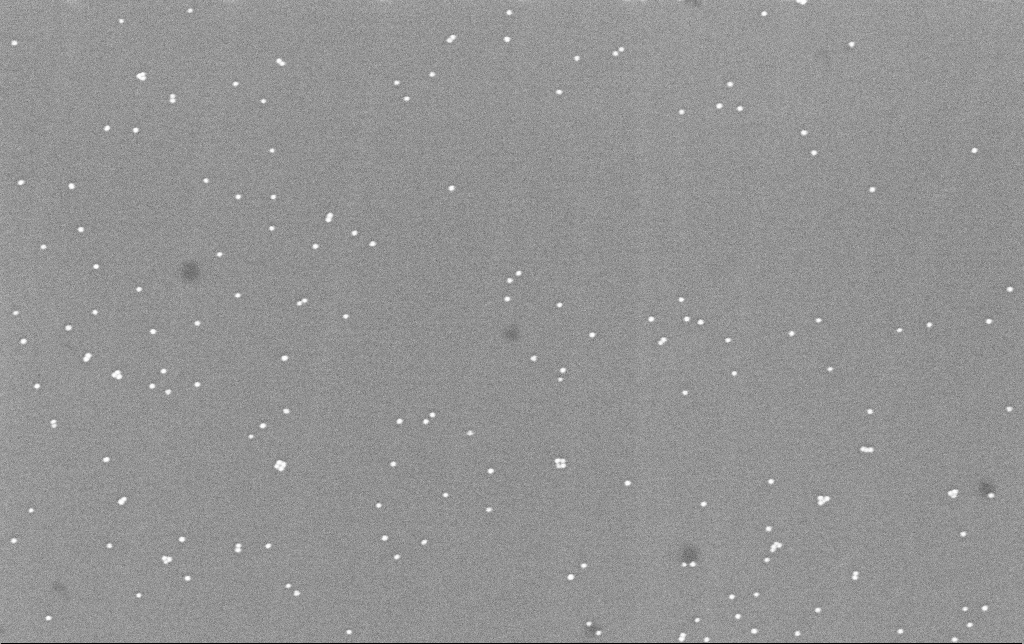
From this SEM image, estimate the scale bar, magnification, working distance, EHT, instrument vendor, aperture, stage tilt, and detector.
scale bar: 200 nm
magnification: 100 K X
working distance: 6.6 mm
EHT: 8 kV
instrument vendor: Zeiss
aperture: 30 µm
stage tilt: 0°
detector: InLens